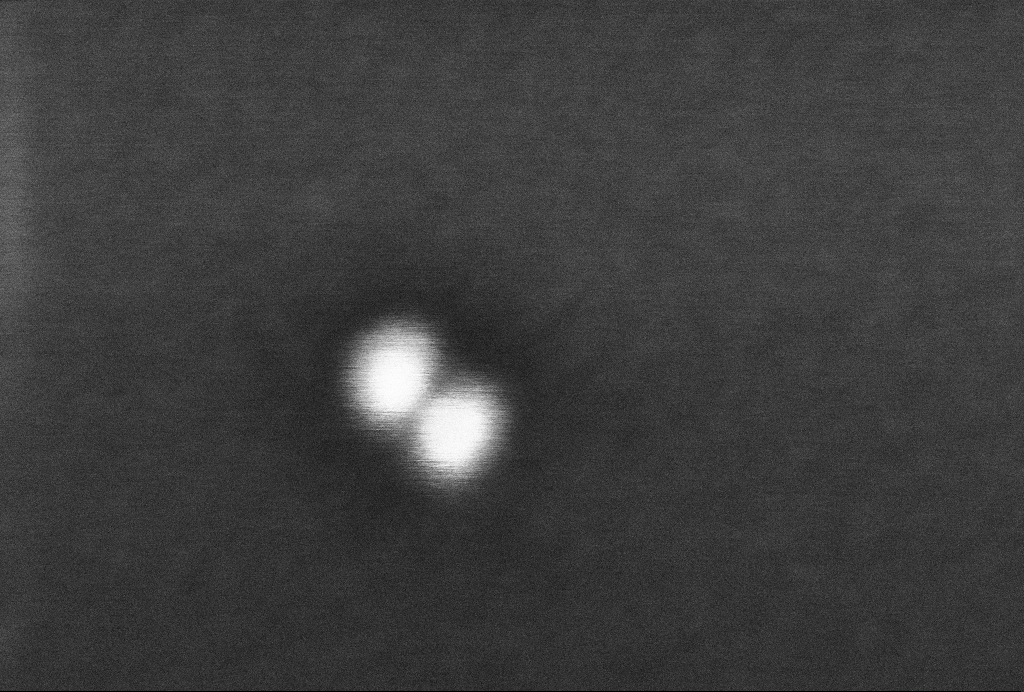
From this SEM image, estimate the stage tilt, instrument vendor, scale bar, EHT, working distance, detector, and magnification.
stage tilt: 0°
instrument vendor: Zeiss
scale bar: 20 nm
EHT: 2 kV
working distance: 3.3 mm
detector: InLens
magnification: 1069.94 K X